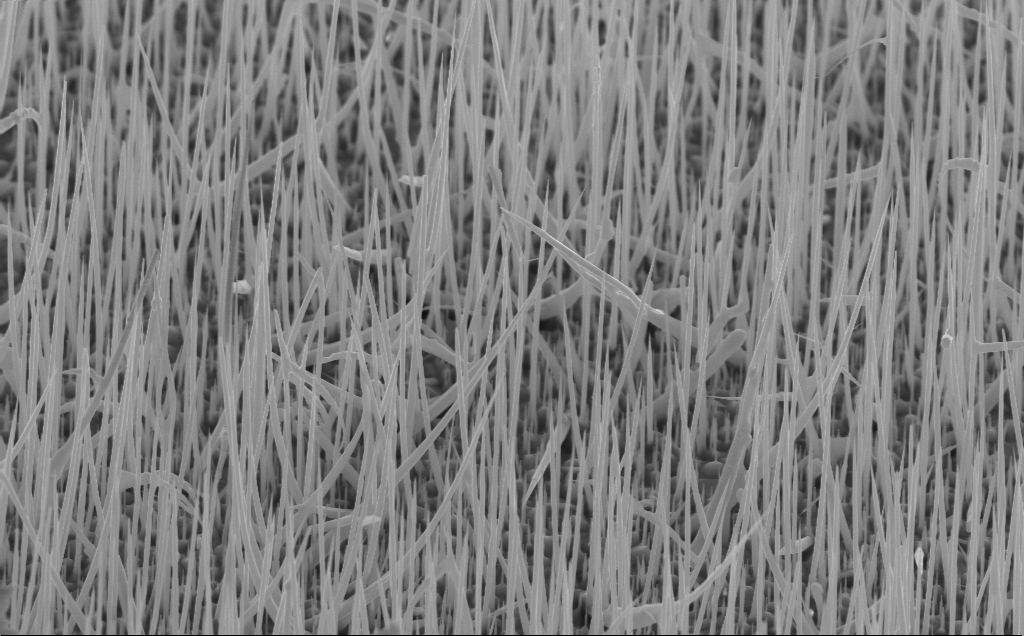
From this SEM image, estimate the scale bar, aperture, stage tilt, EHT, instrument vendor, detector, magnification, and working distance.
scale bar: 1000 nm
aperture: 30 µm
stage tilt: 45°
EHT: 10 kV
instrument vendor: Zeiss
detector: InLens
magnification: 16.76 K X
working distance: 4 mm